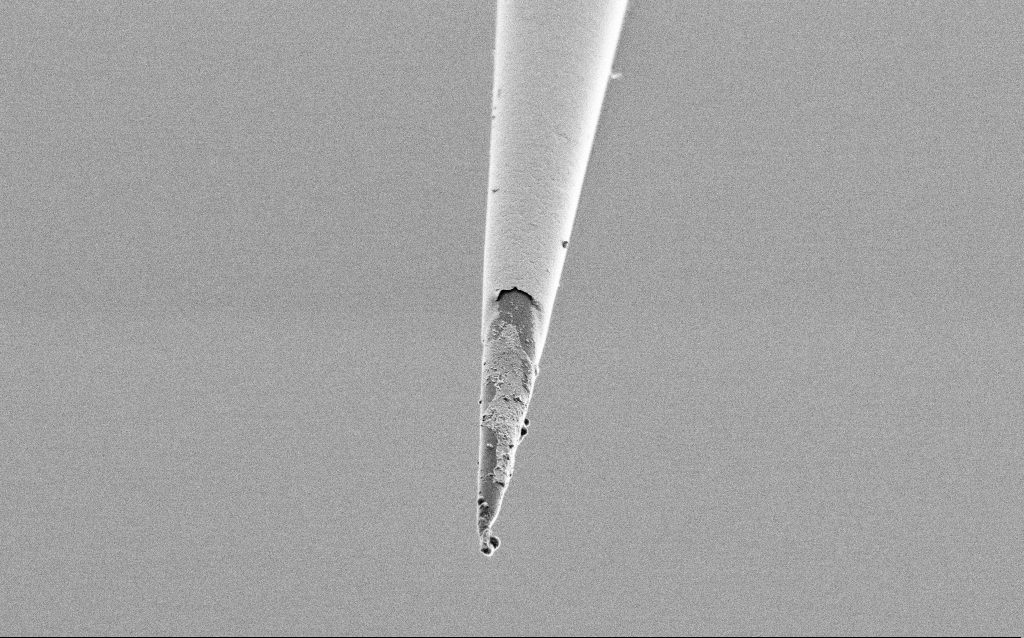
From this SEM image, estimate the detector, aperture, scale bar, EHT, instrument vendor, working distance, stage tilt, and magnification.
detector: SE2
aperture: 30 µm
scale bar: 2000 nm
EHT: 1 kV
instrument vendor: Zeiss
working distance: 6.8 mm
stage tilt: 45°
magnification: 10 K X